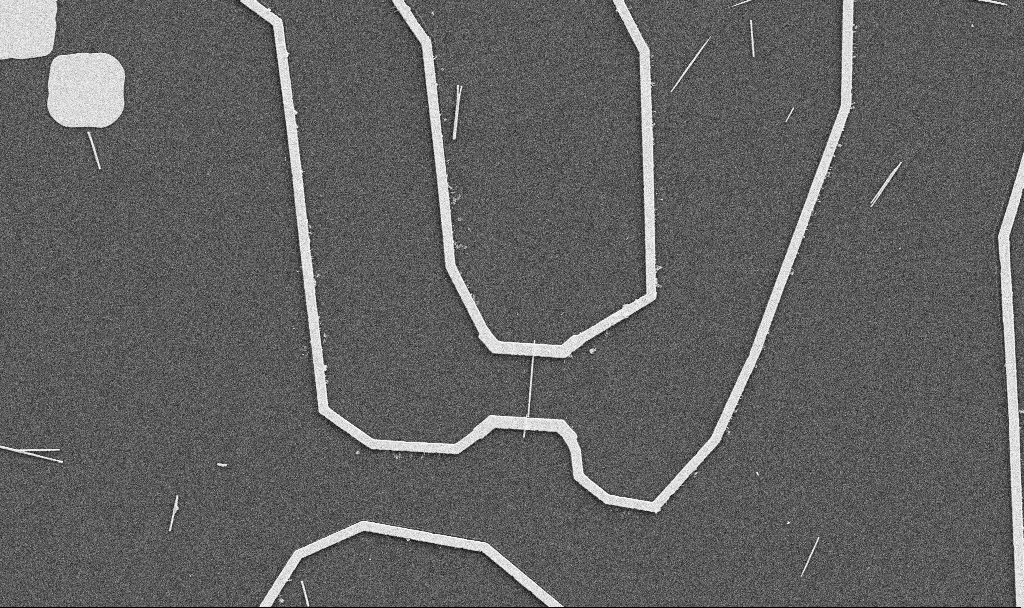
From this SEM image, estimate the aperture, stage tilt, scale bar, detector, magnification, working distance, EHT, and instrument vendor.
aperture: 30 µm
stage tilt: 0°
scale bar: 10000 nm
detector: SE2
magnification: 5 K X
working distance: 10.7 mm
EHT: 5 kV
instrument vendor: Zeiss